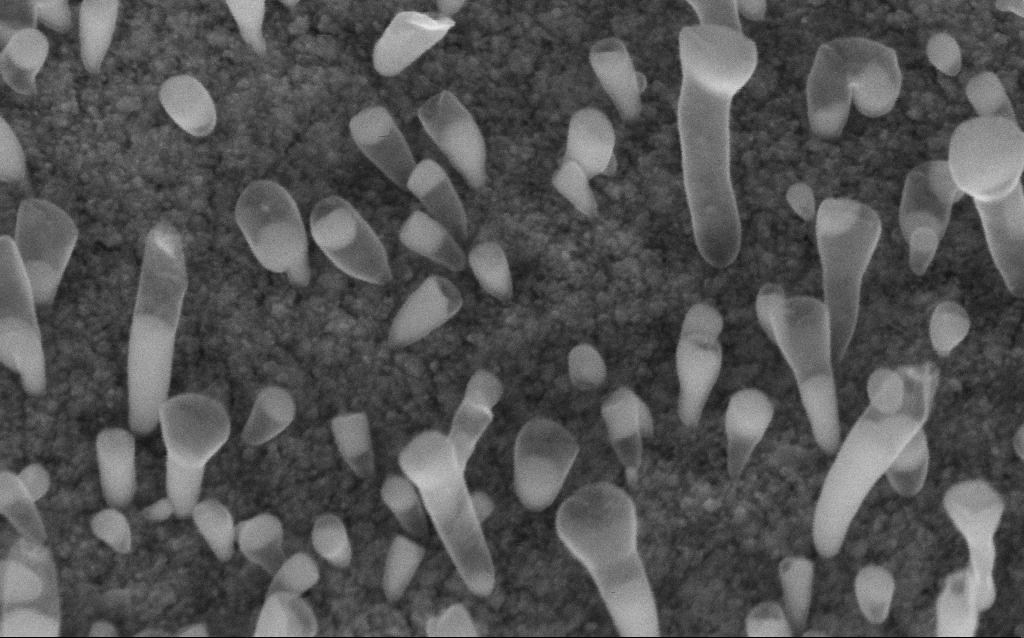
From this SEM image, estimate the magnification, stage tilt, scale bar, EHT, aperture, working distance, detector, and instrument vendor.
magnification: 200 K X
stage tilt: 45°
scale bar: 100 nm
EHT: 5 kV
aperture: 30 µm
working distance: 6.6 mm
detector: InLens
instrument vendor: Zeiss